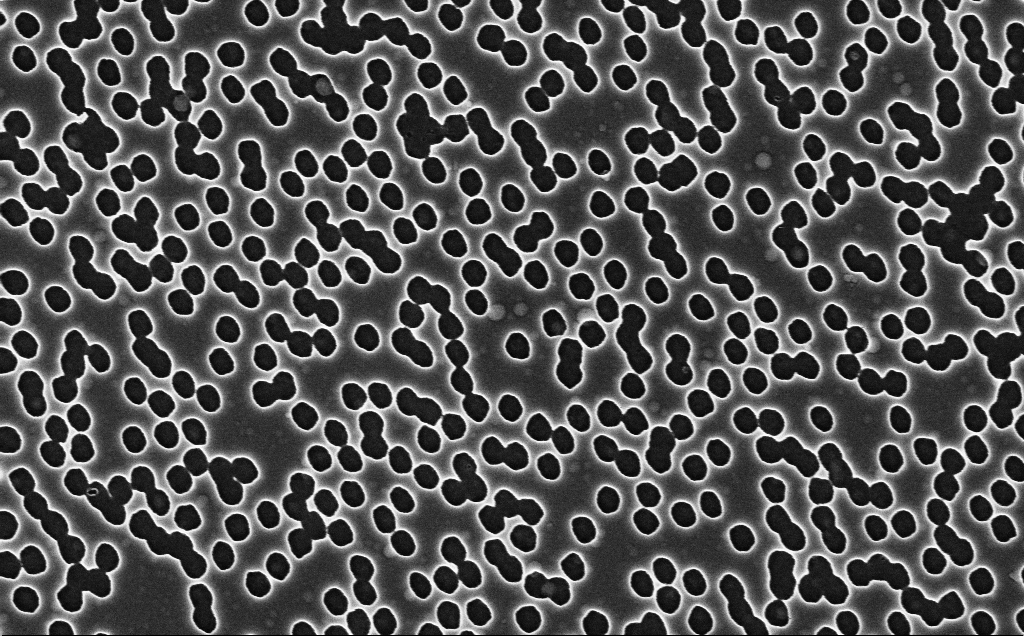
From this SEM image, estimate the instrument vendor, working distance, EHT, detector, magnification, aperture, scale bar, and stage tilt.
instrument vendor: Zeiss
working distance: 2.4 mm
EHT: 3 kV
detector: InLens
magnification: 40 K X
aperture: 30 µm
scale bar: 1000 nm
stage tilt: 0°